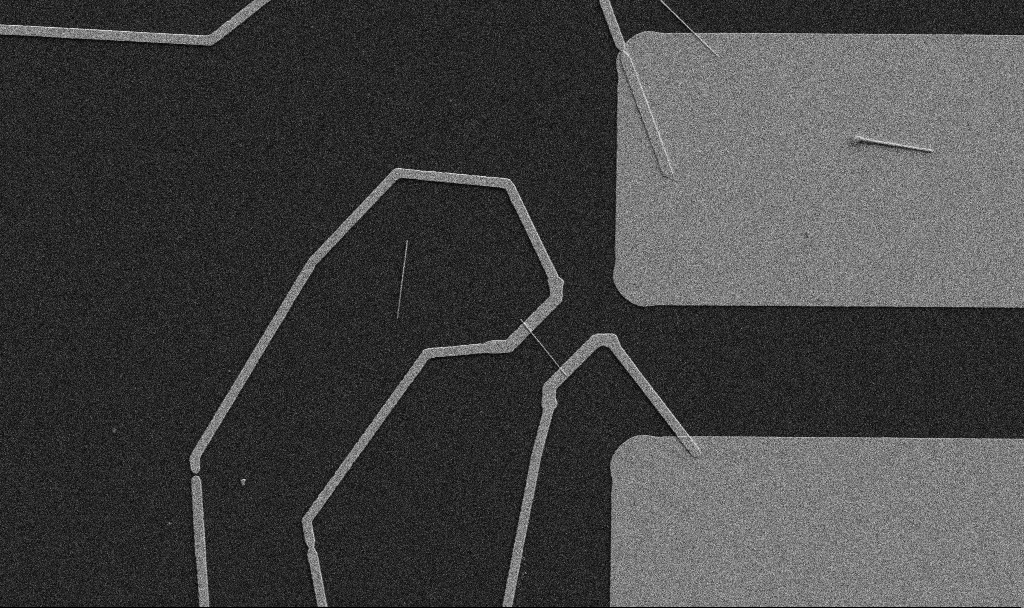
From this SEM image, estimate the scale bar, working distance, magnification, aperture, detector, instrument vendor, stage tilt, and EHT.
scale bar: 10000 nm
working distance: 10.7 mm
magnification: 5 K X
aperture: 30 µm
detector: SE2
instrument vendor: Zeiss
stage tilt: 0°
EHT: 5 kV